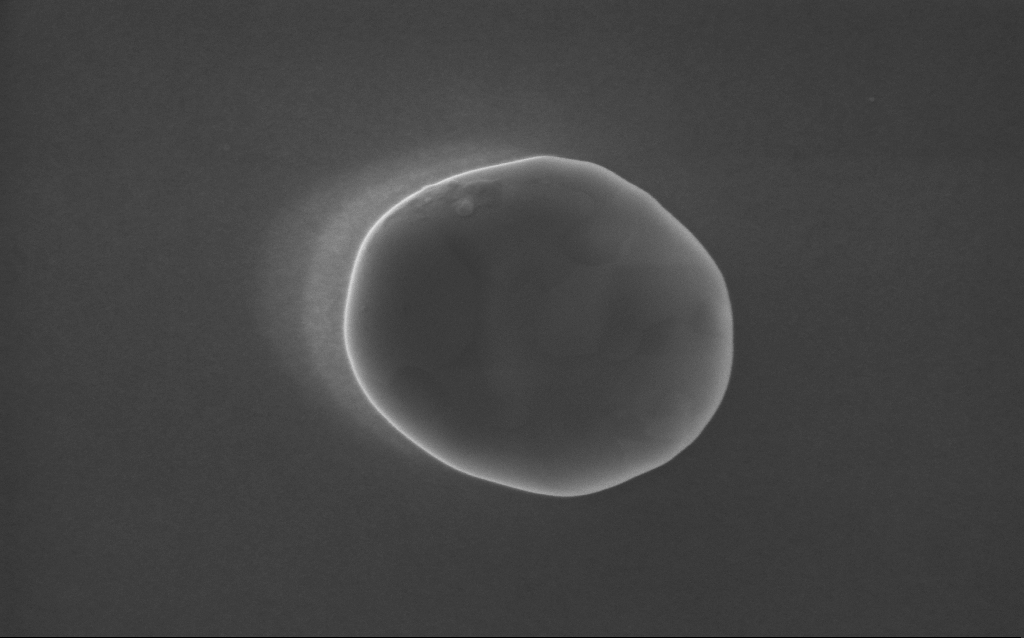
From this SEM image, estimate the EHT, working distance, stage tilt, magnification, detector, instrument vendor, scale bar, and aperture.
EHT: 5 kV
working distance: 3 mm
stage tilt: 0°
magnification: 151 K X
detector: InLens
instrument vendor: Zeiss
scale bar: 100 nm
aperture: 30 µm